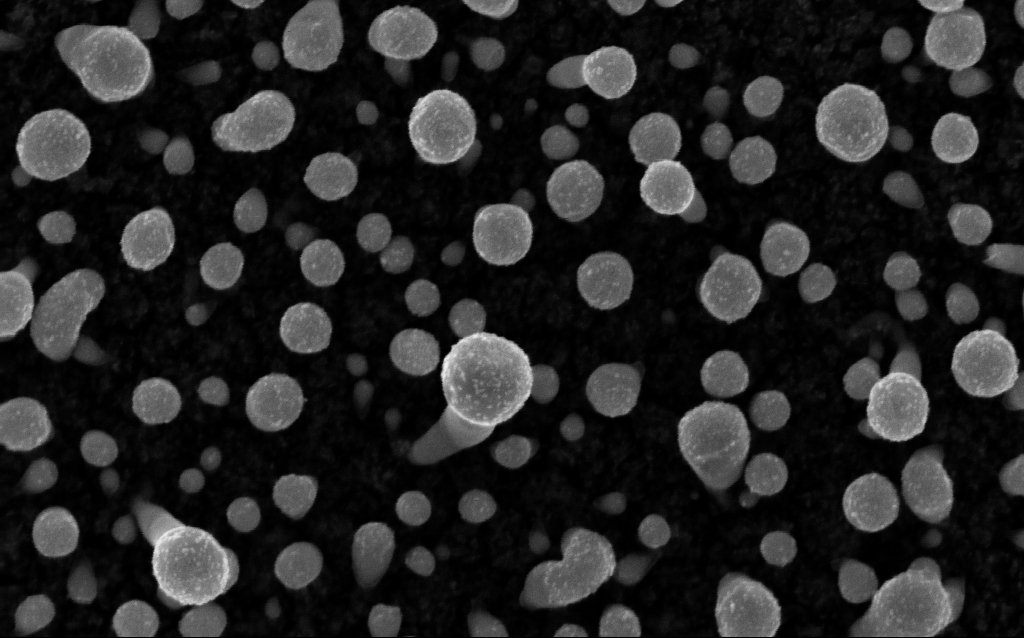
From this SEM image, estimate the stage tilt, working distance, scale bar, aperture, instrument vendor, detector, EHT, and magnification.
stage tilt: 0°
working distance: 2.1 mm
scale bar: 100 nm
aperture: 30 µm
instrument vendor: Zeiss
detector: InLens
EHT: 5 kV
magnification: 200 K X